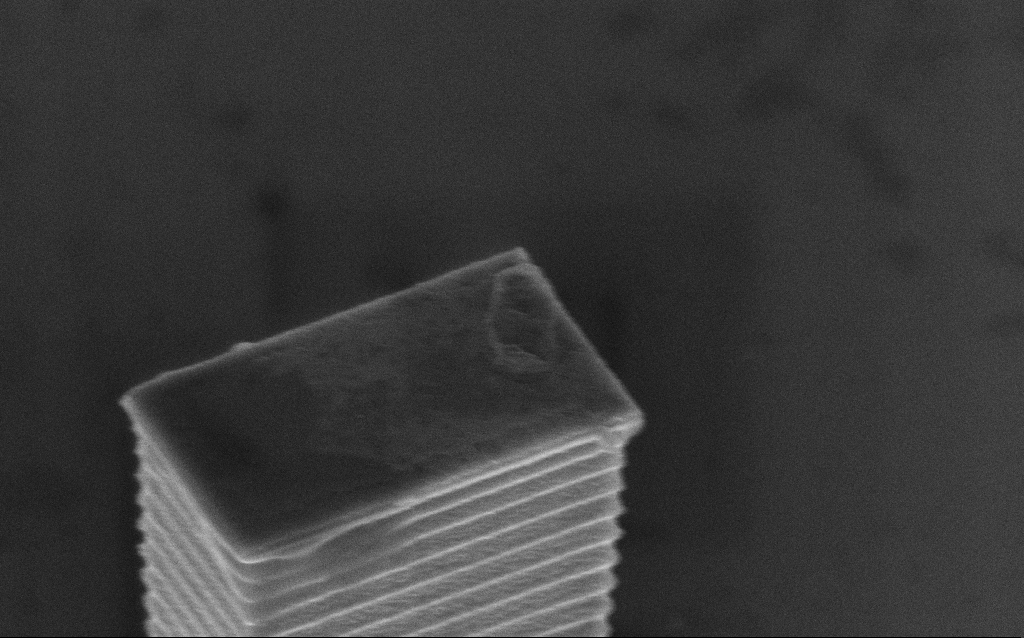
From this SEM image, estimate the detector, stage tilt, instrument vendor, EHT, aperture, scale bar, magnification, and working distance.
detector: InLens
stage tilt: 45°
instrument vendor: Zeiss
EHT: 5 kV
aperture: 30 µm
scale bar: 1000 nm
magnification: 33.8 K X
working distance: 12 mm